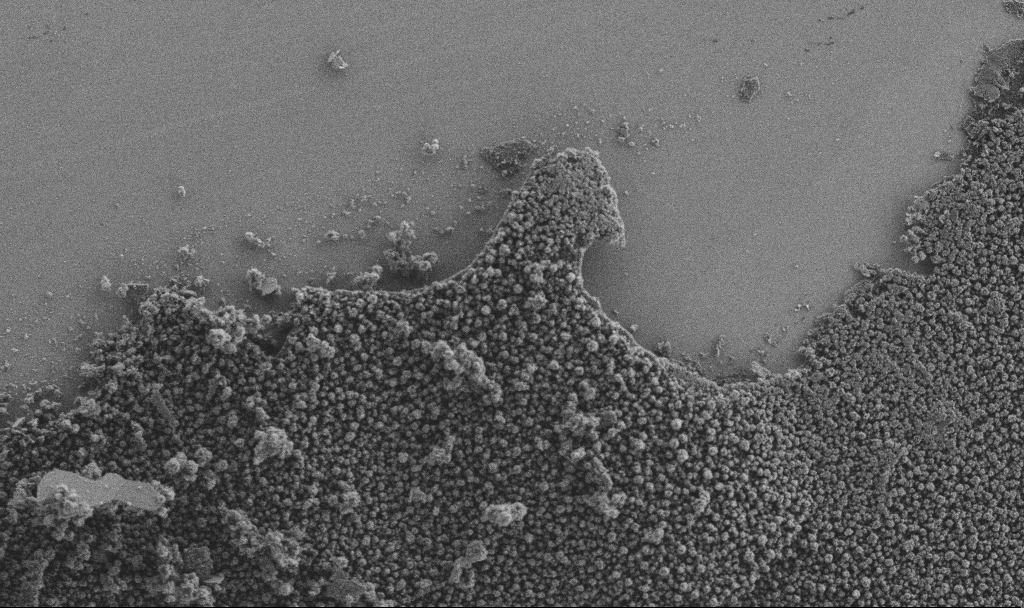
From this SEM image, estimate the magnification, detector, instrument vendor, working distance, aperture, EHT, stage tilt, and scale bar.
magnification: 0.5 K X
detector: SE2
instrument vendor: Zeiss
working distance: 4 mm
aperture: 30 µm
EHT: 3 kV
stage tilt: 0°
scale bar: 100000 nm